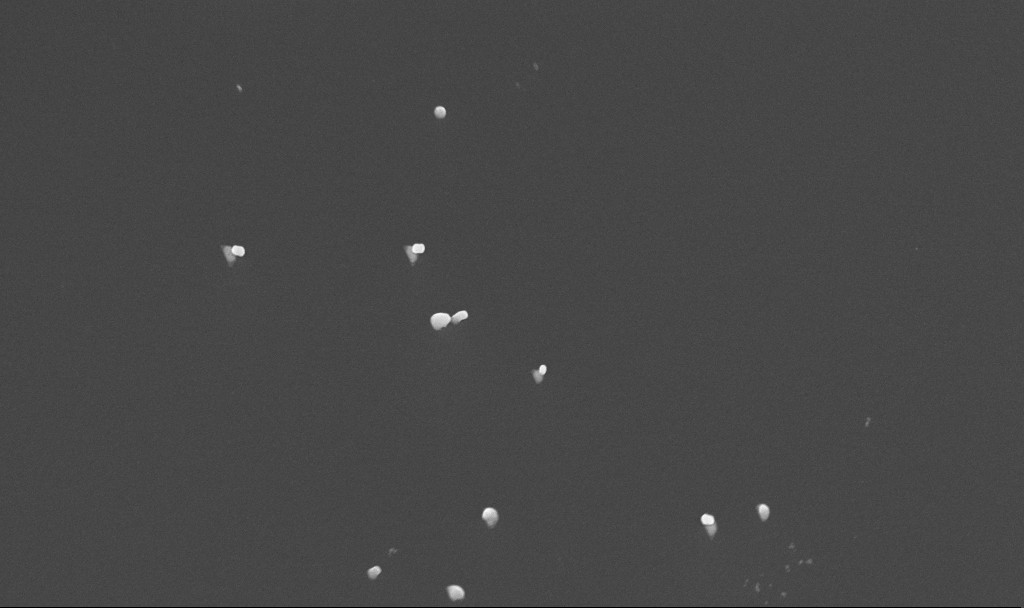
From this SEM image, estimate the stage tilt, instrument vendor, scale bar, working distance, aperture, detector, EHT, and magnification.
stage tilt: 45°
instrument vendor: Zeiss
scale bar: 1000 nm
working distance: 4.8 mm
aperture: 30 µm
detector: InLens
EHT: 10 kV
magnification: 50 K X